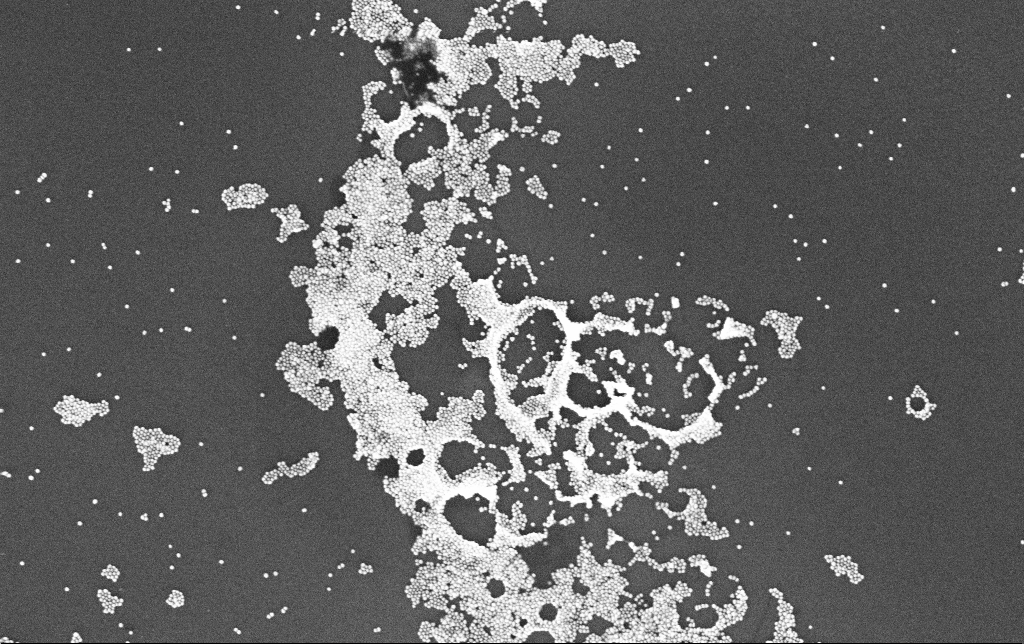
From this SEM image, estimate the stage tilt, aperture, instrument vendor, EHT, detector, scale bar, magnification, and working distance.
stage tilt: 0°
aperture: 30 µm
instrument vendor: Zeiss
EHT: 10 kV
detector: InLens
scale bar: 200 nm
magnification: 100 K X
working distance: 3.4 mm